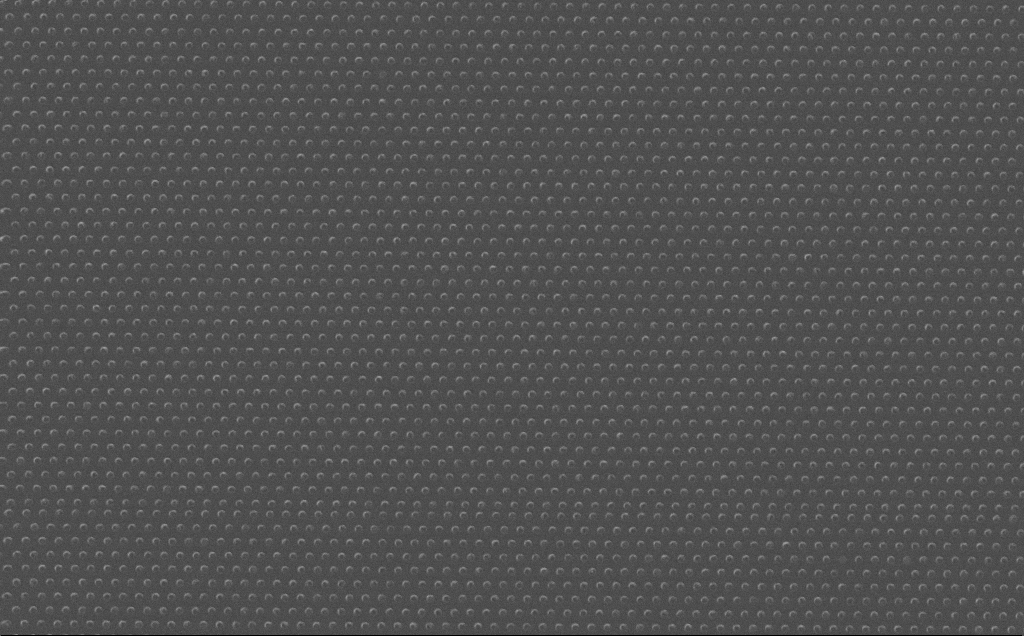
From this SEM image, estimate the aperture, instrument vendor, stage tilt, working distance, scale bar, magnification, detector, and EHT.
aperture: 30 µm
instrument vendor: Zeiss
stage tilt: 0°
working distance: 7 mm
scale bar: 2000 nm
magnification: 16.98 K X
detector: InLens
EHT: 10 kV